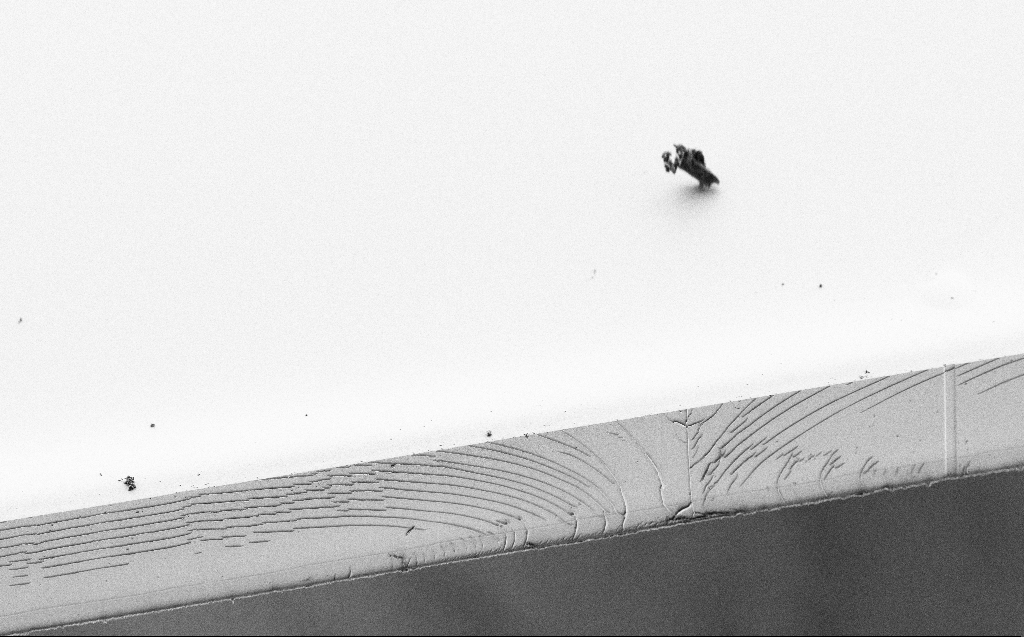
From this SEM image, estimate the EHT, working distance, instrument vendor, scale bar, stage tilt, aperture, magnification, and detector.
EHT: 3 kV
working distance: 4 mm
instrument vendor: Zeiss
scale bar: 100000 nm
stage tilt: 45°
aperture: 30 µm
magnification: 0.534 K X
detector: SE2